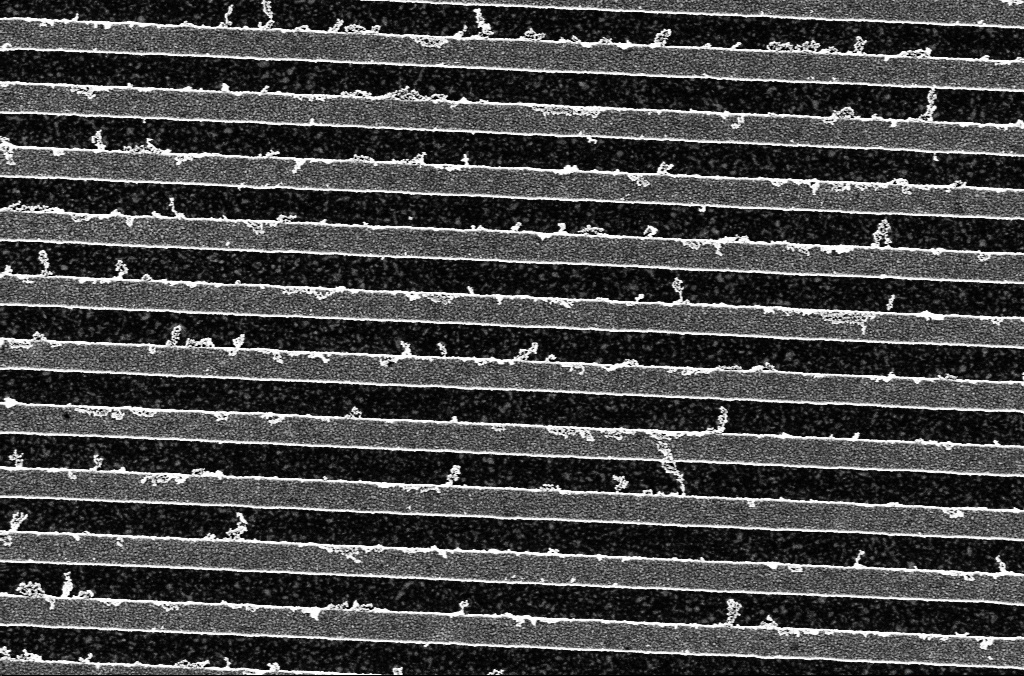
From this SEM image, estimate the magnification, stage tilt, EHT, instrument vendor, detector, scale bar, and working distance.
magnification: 47.13 K X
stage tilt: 0°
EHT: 2 kV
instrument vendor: Zeiss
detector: InLens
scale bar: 1000 nm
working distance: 3.9 mm